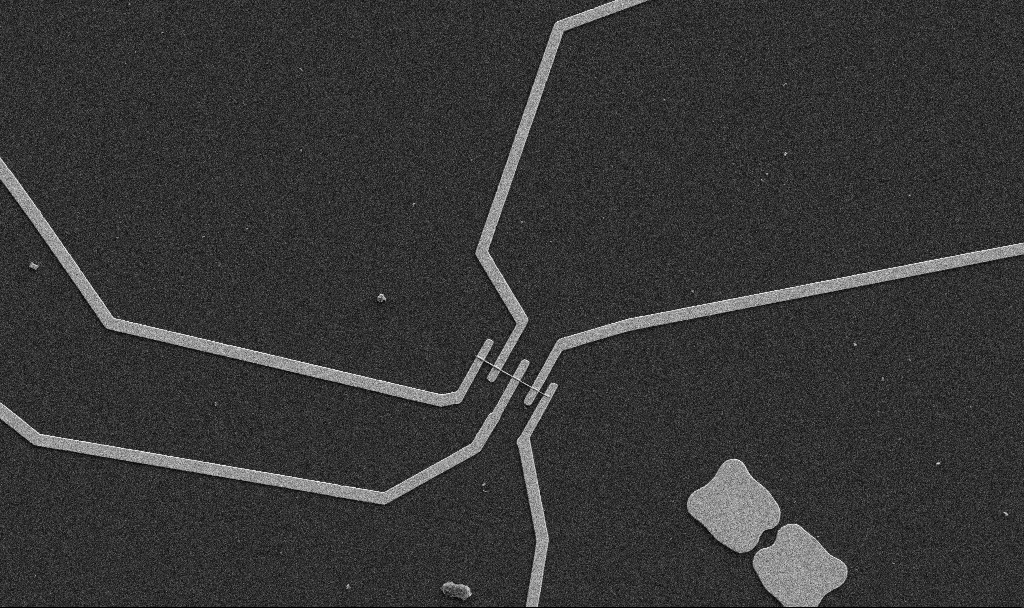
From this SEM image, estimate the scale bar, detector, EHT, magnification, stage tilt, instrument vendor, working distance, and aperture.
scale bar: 10000 nm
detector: SE2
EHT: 5 kV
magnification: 5 K X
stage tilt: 0°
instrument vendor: Zeiss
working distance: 10.7 mm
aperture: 30 µm